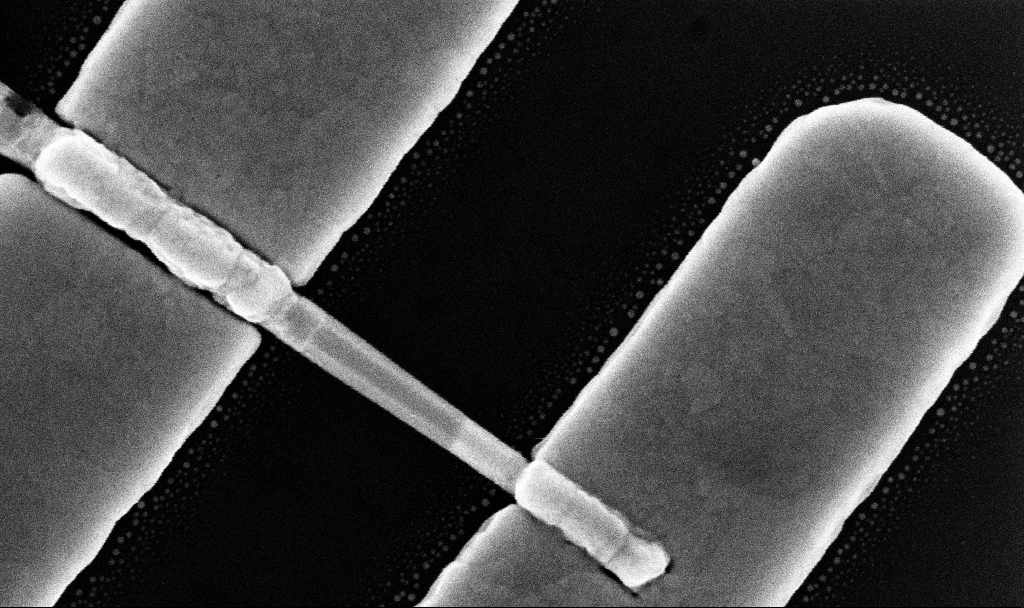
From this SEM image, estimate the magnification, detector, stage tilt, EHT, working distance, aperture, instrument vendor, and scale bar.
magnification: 166.22 K X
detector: InLens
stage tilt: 0°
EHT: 10 kV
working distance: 7.7 mm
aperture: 30 µm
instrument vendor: Zeiss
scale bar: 200 nm